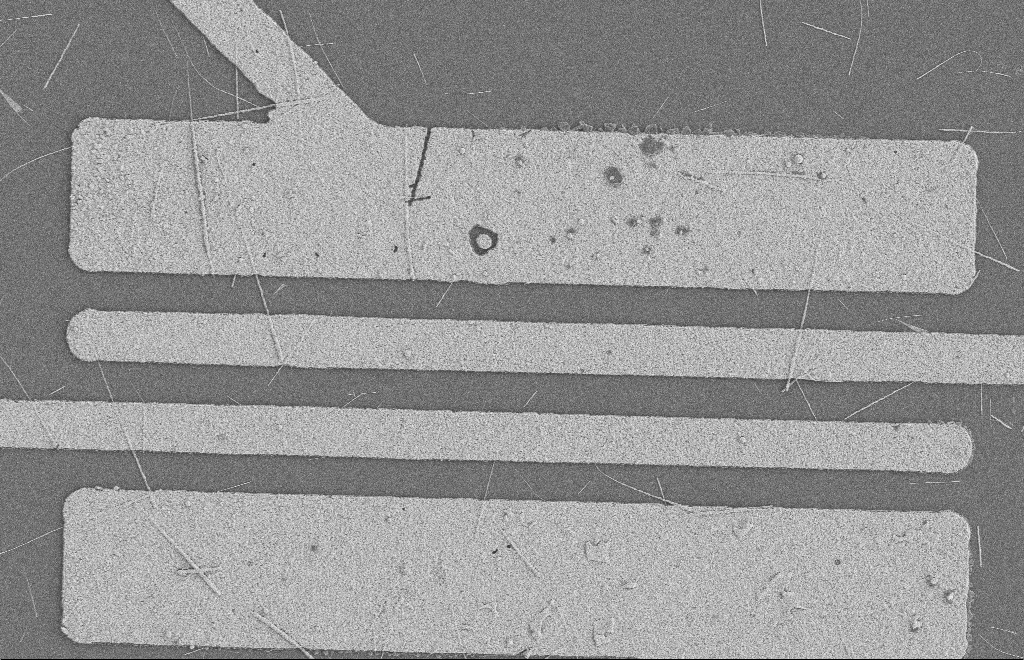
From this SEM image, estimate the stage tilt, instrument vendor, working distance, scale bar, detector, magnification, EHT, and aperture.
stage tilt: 0°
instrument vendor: Zeiss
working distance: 8 mm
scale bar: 2000 nm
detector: SE2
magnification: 5.46 K X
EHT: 2 kV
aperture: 20 µm